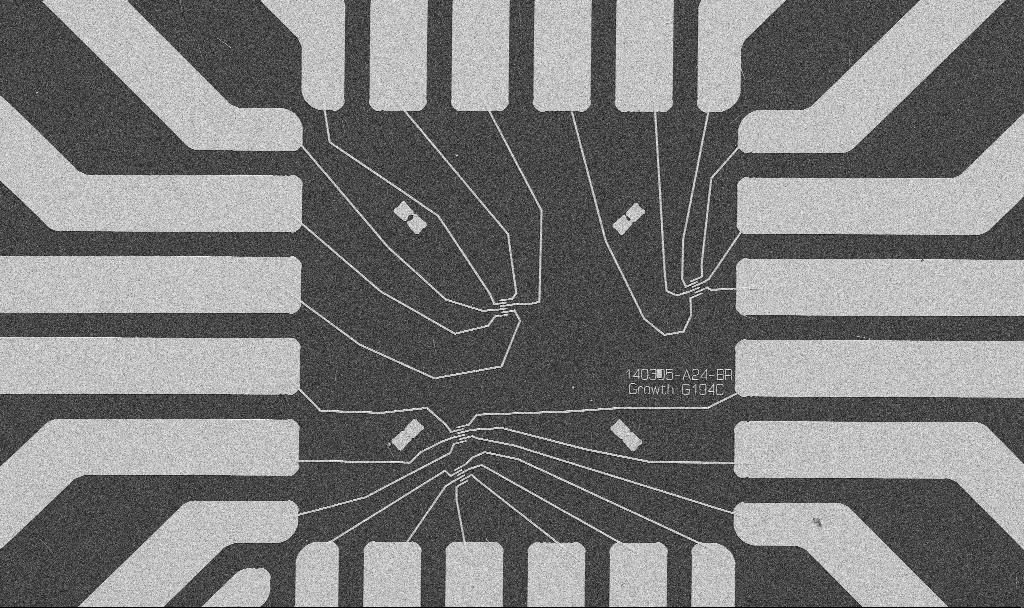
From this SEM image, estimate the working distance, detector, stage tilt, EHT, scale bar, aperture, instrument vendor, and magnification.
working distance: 10.7 mm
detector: SE2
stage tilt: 0°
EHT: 5 kV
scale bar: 20000 nm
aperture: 30 µm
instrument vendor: Zeiss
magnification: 1 K X